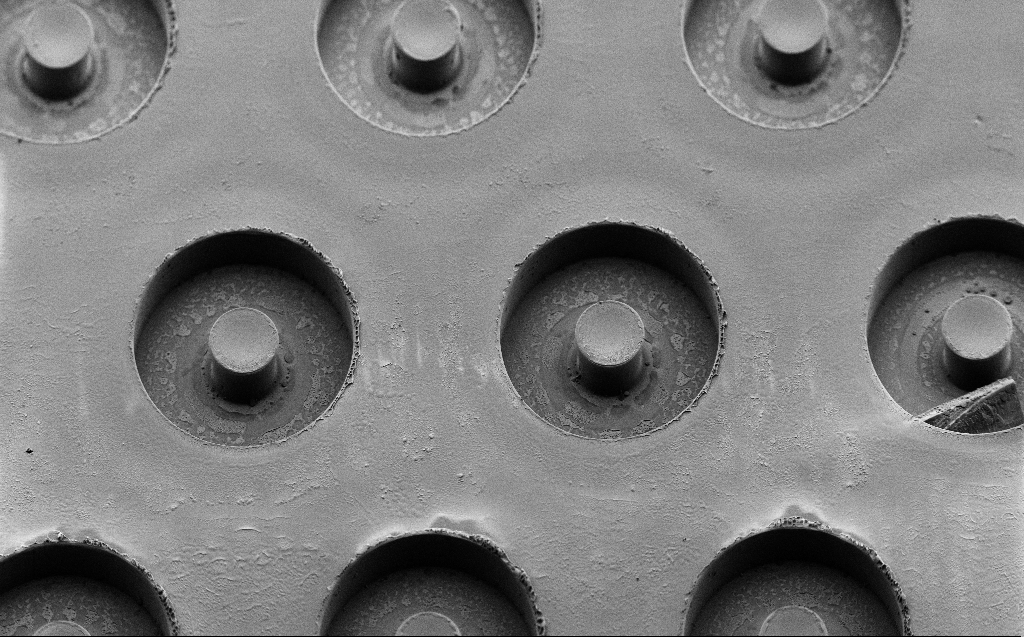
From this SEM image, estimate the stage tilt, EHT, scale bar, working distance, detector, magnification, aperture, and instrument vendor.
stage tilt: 45°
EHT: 2 kV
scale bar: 100000 nm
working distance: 7 mm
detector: SE2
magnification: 0.622 K X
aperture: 30 µm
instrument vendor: Zeiss